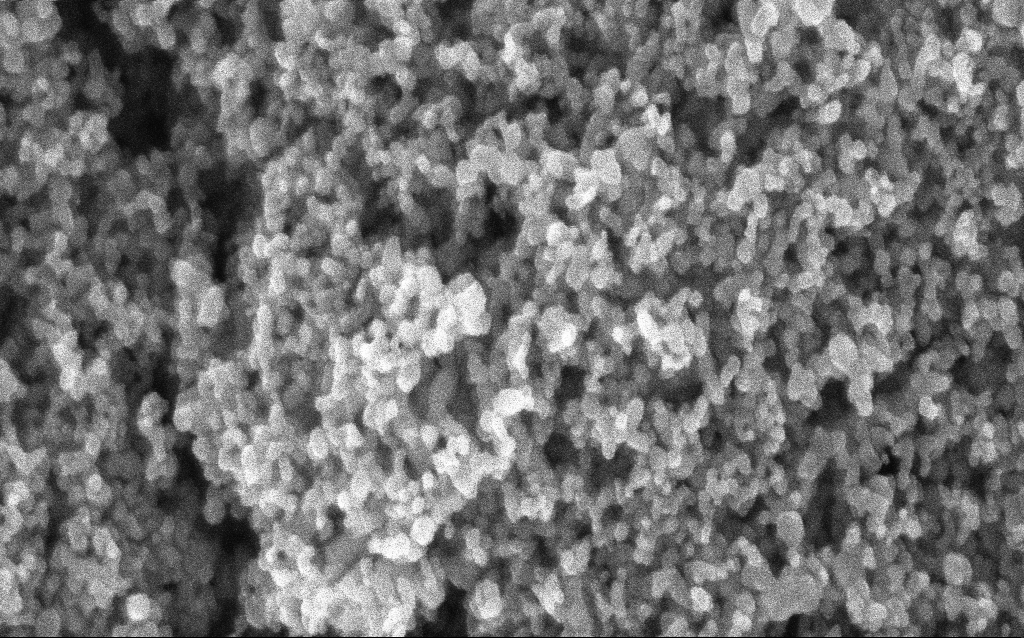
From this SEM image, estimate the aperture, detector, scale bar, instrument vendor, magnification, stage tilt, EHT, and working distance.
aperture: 30 µm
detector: InLens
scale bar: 100 nm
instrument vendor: Zeiss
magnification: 204.13 K X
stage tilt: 0°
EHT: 5 kV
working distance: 2.7 mm